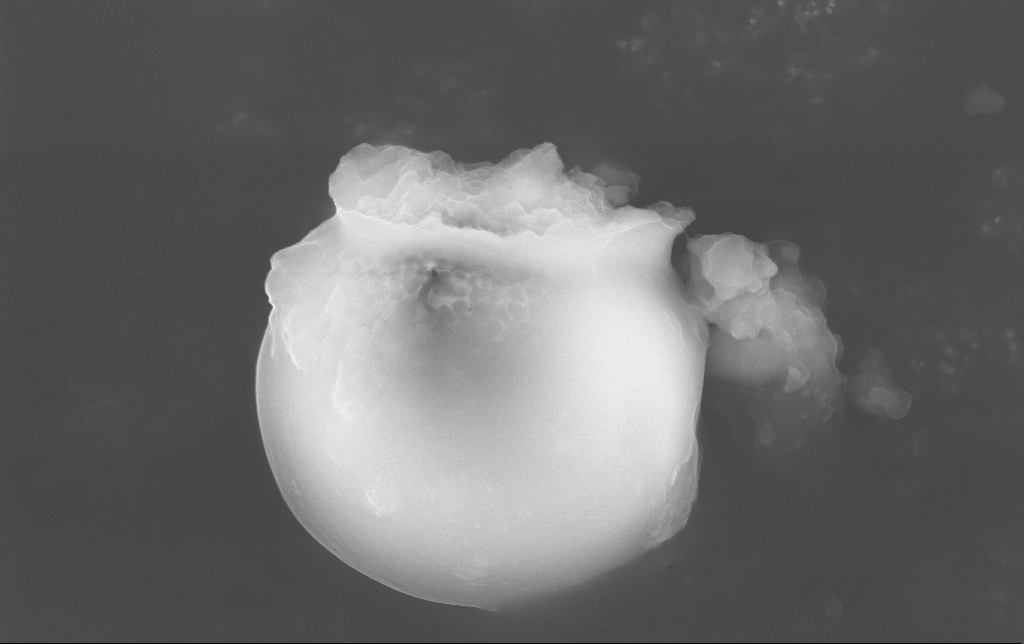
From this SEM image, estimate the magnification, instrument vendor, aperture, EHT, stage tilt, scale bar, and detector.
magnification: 28.13 K X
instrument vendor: Zeiss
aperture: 30 µm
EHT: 15 kV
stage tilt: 0°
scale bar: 2000 nm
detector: InLens